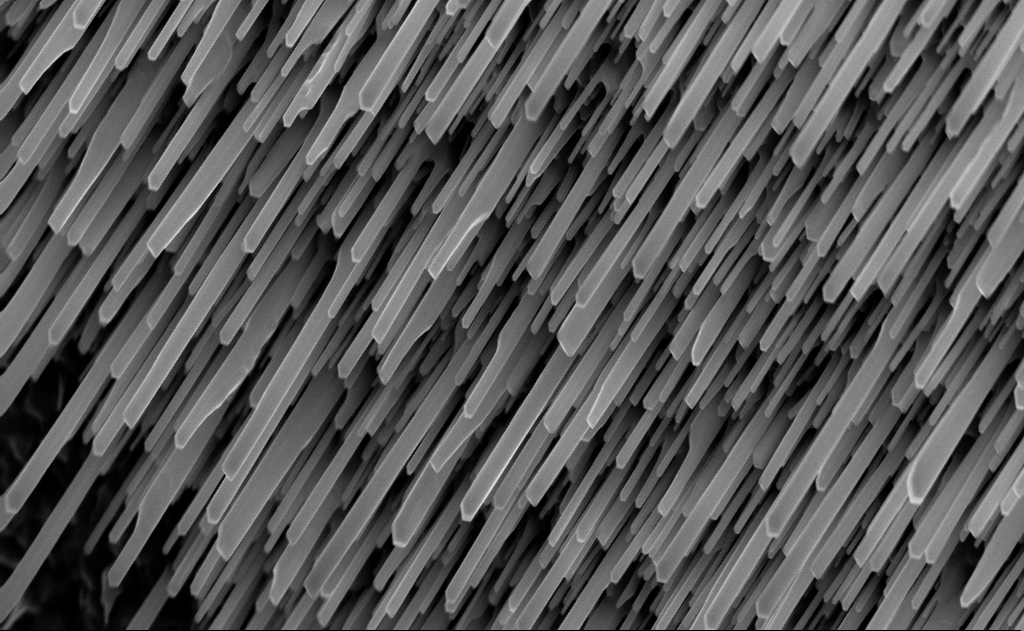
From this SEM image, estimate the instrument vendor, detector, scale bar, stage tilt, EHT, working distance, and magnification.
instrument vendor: Zeiss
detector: InLens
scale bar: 1000 nm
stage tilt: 0°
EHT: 10 kV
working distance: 7 mm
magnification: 40 K X